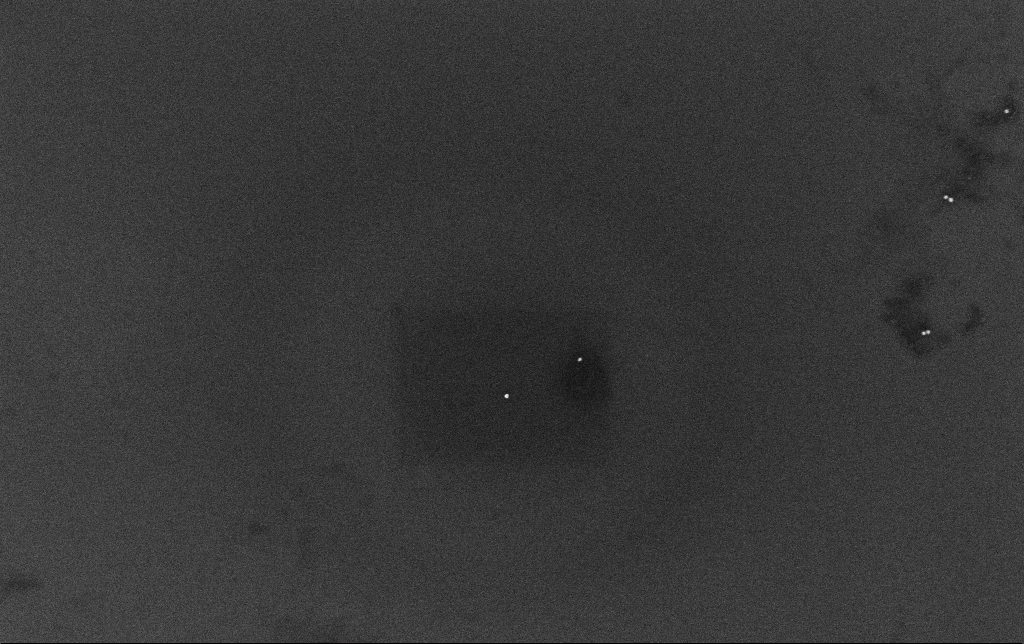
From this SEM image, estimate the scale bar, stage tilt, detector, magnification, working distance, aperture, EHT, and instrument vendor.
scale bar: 200 nm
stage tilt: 0°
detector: InLens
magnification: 100 K X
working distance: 3.4 mm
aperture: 30 µm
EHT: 10 kV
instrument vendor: Zeiss